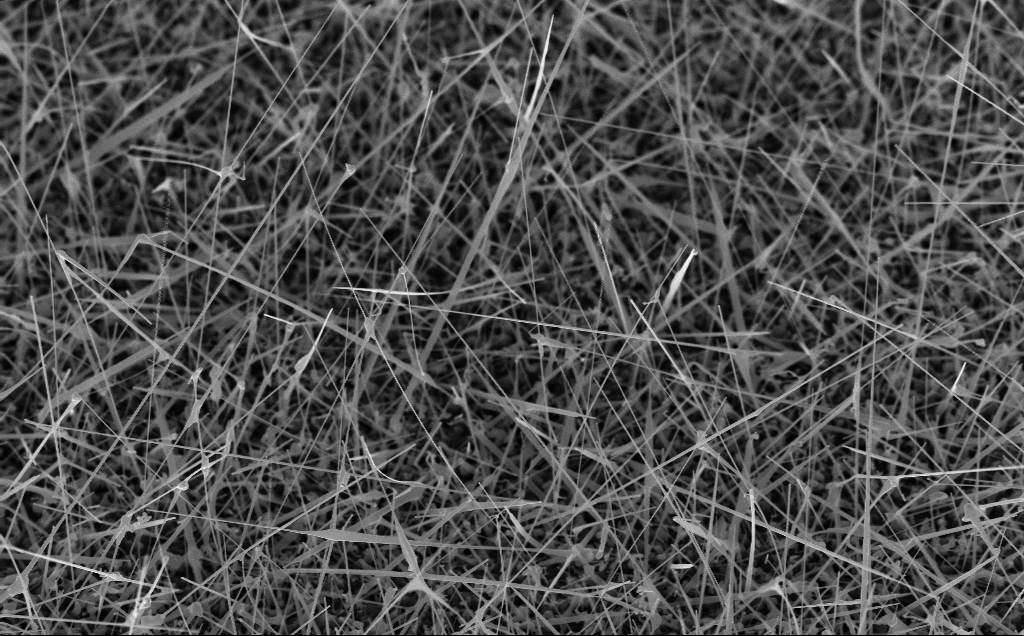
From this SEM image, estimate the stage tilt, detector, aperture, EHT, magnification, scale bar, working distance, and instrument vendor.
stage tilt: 45°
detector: InLens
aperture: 30 µm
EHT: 10 kV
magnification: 20 K X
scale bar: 1000 nm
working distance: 8 mm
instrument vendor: Zeiss